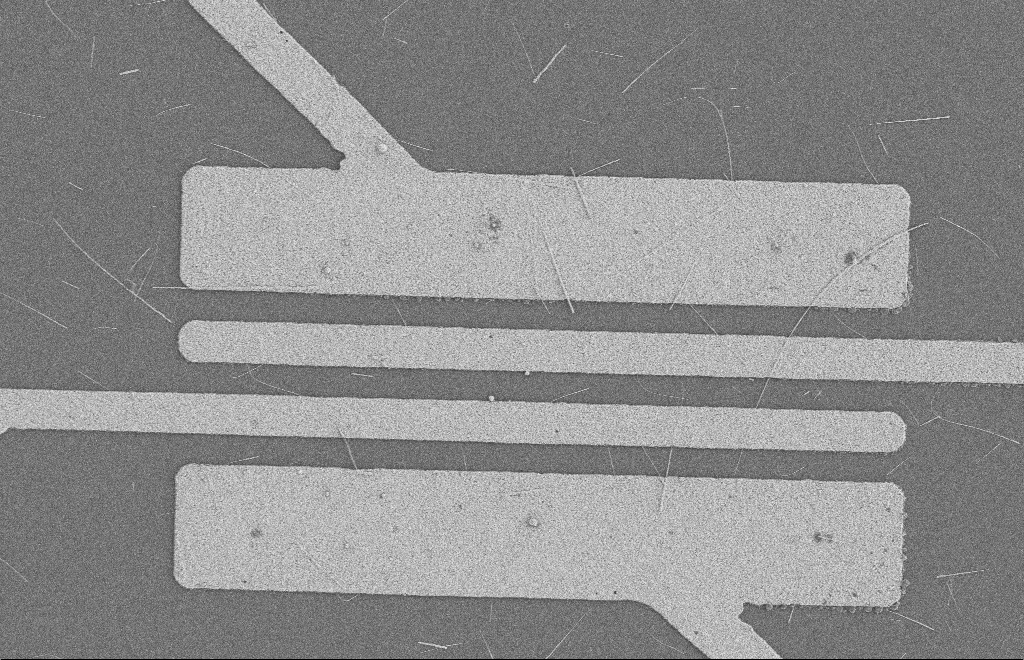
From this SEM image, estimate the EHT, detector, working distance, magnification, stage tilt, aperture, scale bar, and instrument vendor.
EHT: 2 kV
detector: SE2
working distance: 8 mm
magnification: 4.39 K X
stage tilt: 0°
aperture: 20 µm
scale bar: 10000 nm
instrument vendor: Zeiss